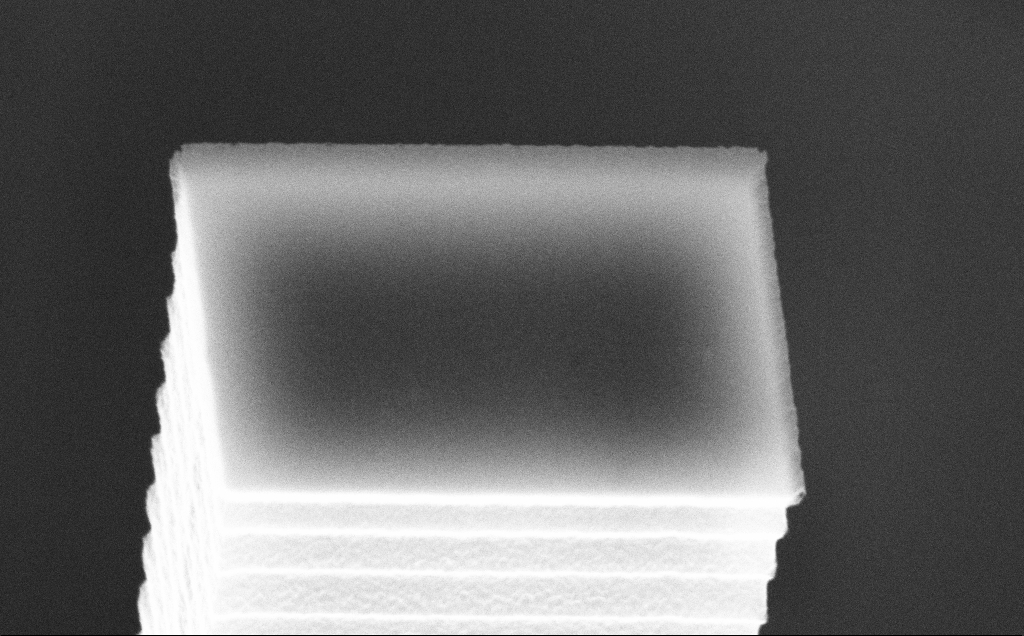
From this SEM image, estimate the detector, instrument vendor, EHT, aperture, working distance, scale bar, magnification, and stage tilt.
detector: InLens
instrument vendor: Zeiss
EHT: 10 kV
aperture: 30 µm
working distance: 7 mm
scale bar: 1000 nm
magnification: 46.08 K X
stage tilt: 45°